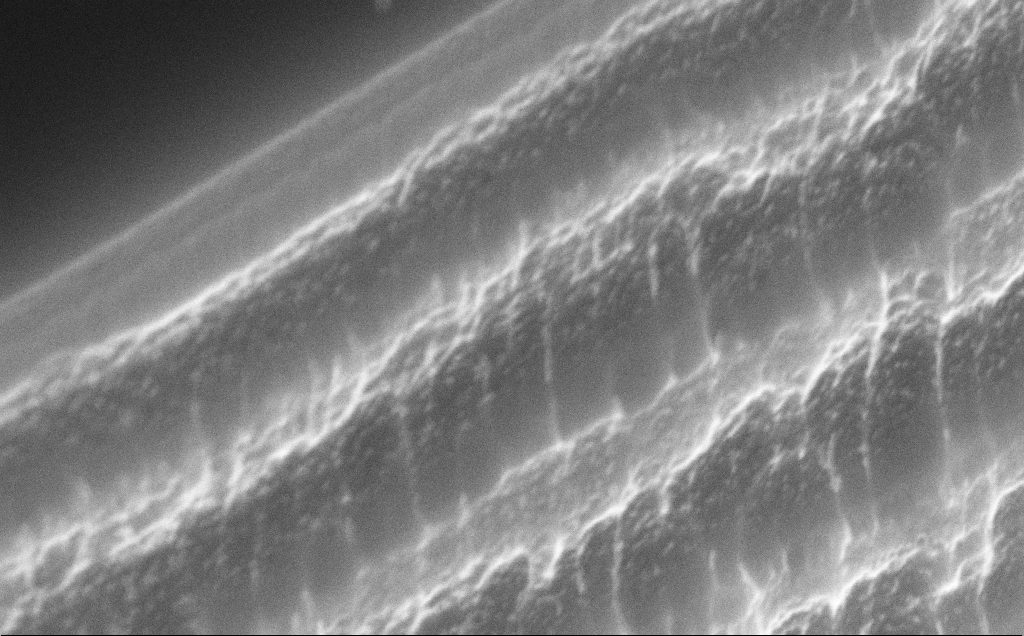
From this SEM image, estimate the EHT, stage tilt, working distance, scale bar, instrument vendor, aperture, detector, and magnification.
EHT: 10 kV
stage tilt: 50°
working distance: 11 mm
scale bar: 200 nm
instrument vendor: Zeiss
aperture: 30 µm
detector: InLens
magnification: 86.44 K X